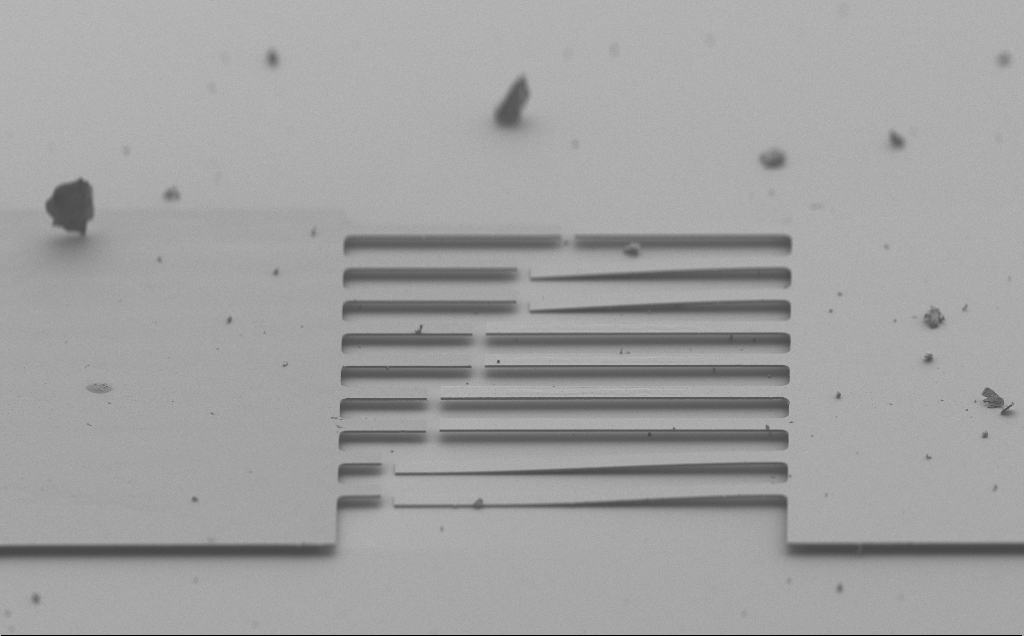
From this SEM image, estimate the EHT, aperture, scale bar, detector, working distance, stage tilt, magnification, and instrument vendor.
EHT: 3 kV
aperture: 30 µm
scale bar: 100000 nm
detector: SE2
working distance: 5 mm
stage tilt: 70°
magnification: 0.651 K X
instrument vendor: Zeiss